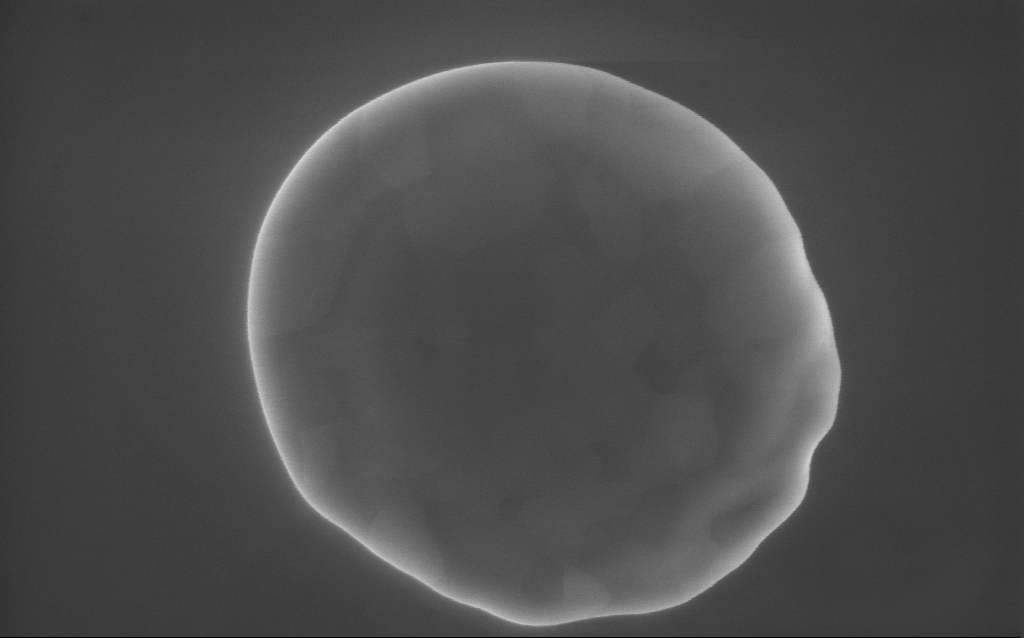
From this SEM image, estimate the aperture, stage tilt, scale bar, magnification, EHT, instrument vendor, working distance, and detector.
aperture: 30 µm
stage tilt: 0°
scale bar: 200 nm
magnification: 90 K X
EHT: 10 kV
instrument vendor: Zeiss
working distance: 3 mm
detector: InLens